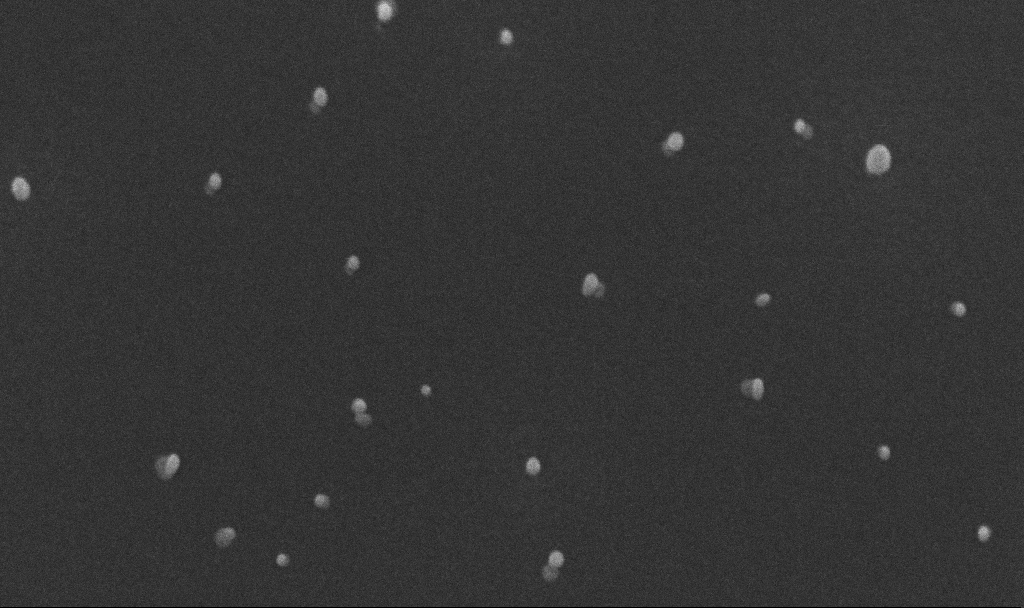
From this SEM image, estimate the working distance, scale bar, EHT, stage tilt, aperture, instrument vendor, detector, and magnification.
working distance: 4.8 mm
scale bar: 200 nm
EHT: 10 kV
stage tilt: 45°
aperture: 30 µm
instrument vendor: Zeiss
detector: InLens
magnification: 200 K X